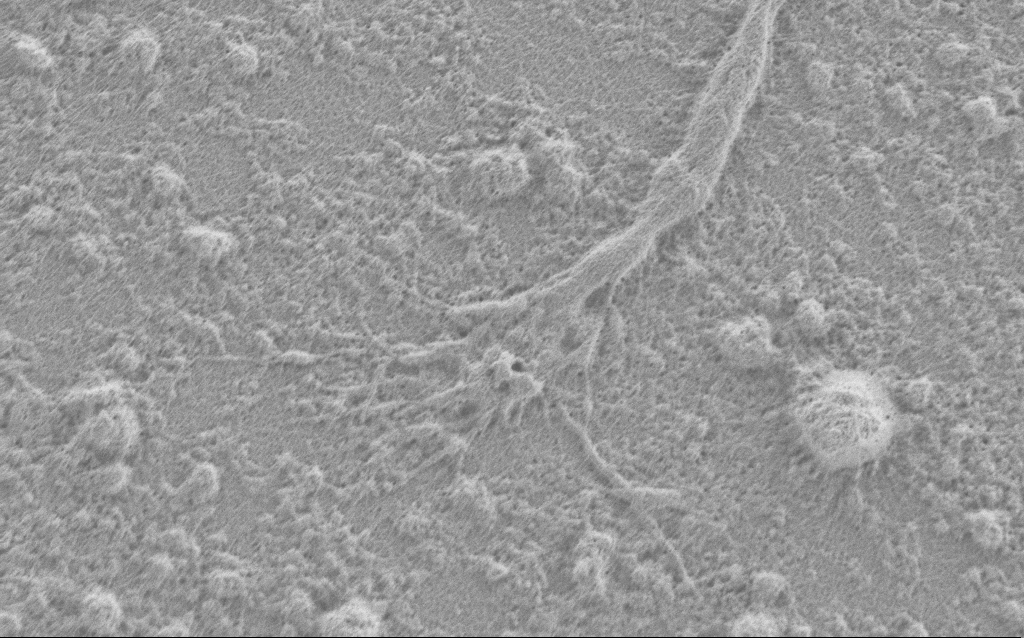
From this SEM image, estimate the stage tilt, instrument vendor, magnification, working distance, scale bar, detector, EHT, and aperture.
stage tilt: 0°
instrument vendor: Zeiss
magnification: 10 K X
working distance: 4 mm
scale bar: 2000 nm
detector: SE2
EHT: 0.9 kV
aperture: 30 µm